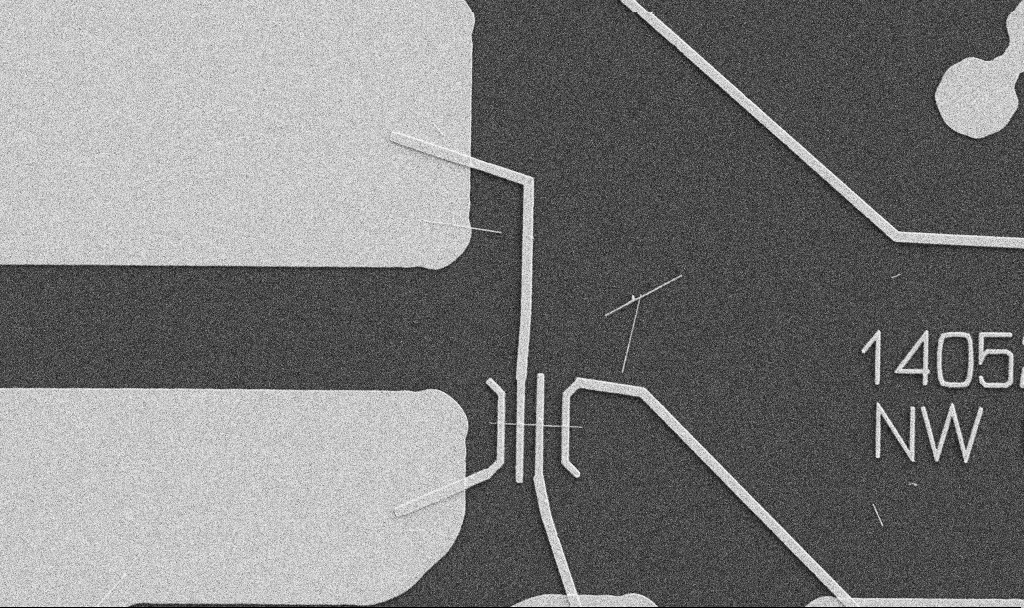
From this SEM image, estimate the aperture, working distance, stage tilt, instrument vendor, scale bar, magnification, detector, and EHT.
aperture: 30 µm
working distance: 10.7 mm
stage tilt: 0°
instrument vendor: Zeiss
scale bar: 10000 nm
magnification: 5 K X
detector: SE2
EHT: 5 kV